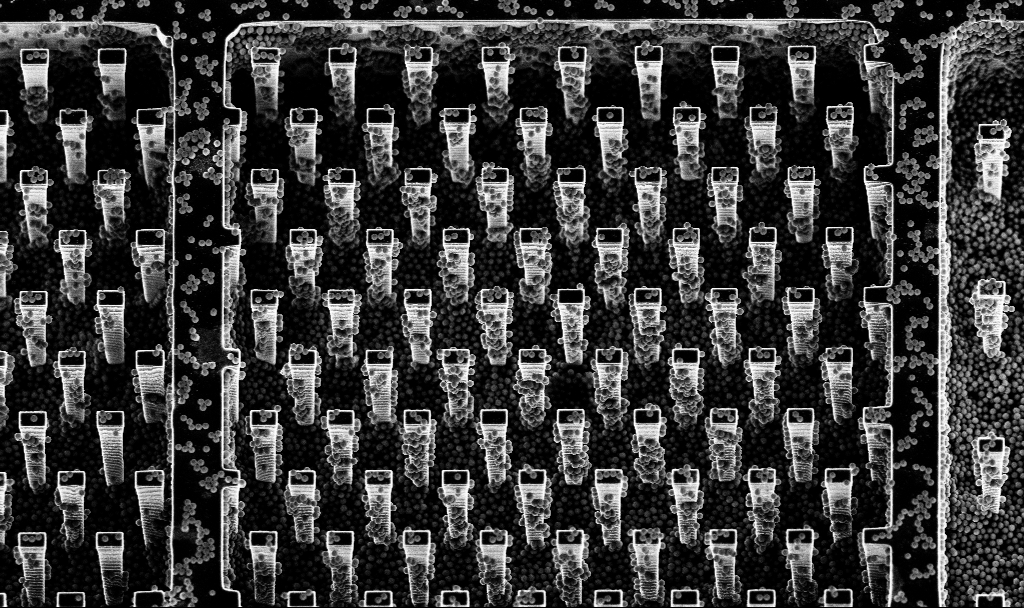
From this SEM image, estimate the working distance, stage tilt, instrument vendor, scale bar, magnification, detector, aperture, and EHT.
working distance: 4.6 mm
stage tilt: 20°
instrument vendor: Zeiss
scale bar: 10000 nm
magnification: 4.34 K X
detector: InLens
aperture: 30 µm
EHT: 5 kV